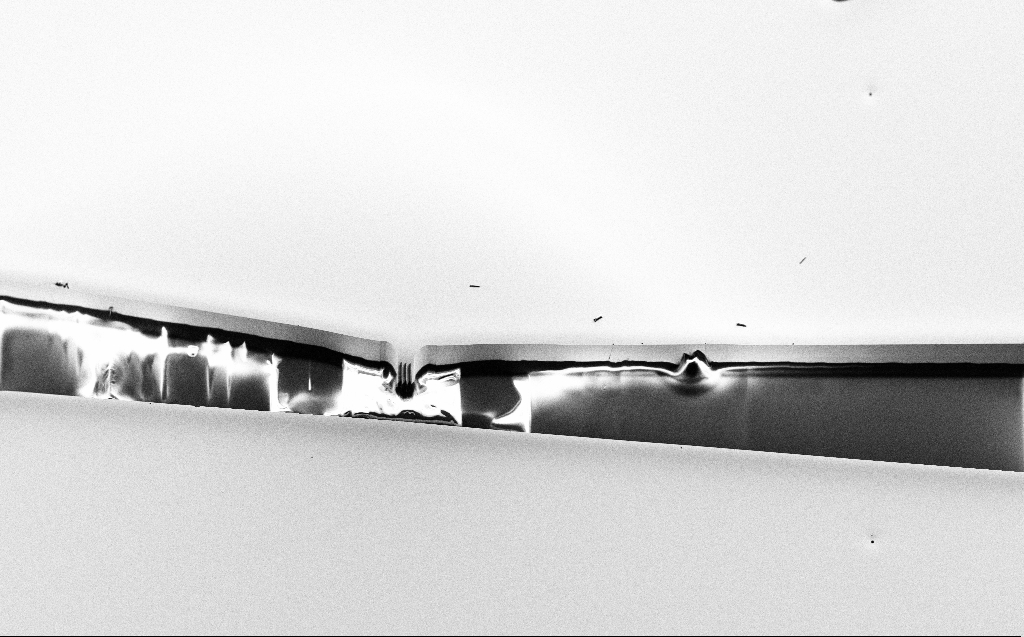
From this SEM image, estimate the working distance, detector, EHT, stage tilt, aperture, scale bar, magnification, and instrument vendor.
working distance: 7 mm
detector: SE2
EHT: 10 kV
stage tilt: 45°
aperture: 30 µm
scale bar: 100000 nm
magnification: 0.144 K X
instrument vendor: Zeiss